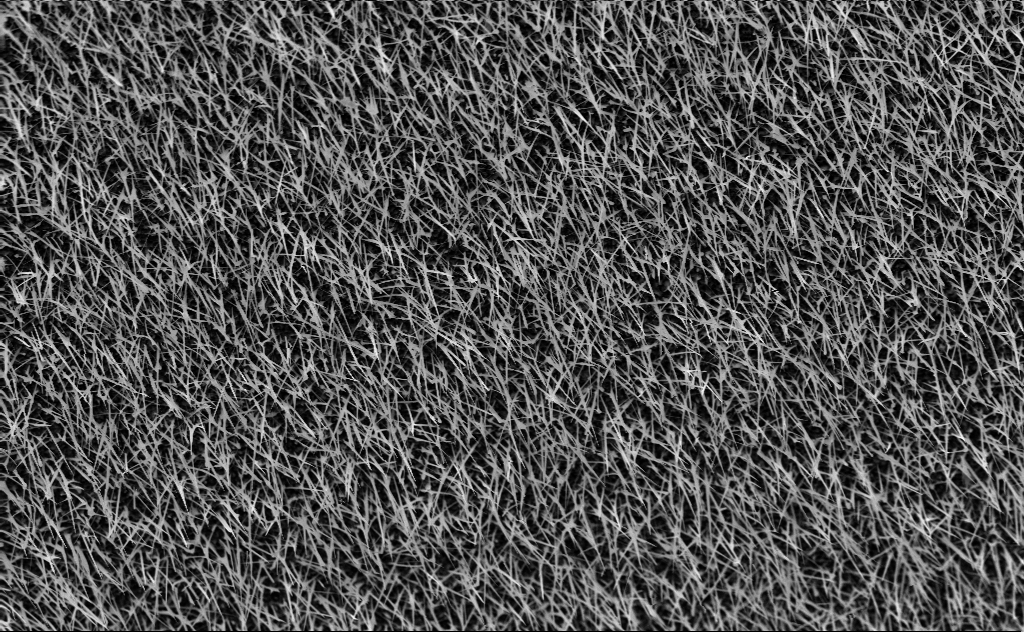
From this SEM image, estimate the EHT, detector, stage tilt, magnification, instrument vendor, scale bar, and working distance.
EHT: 10 kV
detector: InLens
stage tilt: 45°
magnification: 10 K X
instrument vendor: Zeiss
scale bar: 2000 nm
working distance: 5 mm